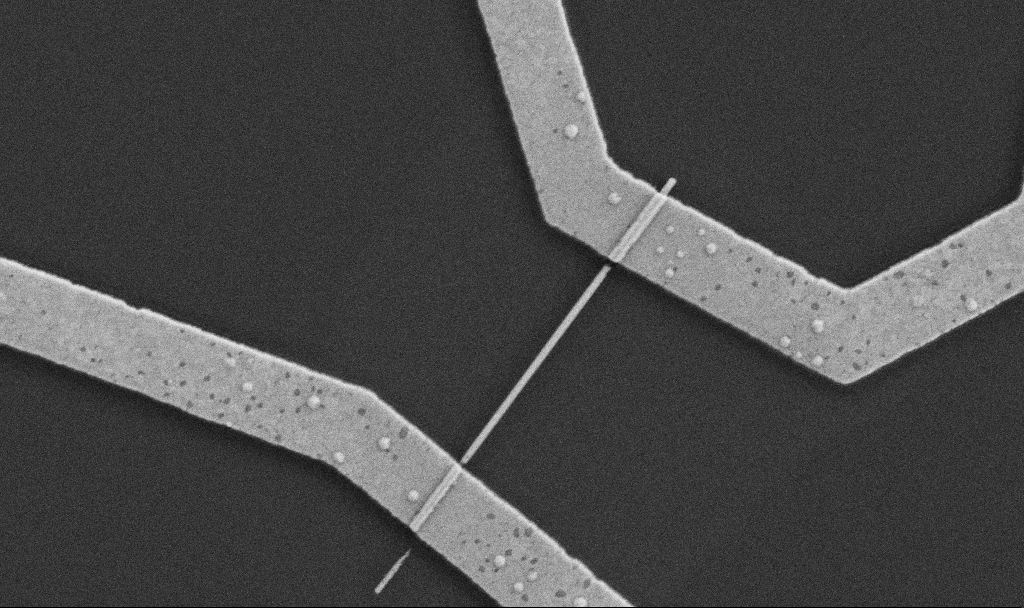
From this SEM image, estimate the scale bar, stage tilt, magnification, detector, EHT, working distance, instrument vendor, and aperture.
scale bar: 2000 nm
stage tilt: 0°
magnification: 30 K X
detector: SE2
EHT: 5 kV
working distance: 7.6 mm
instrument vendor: Zeiss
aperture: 30 µm